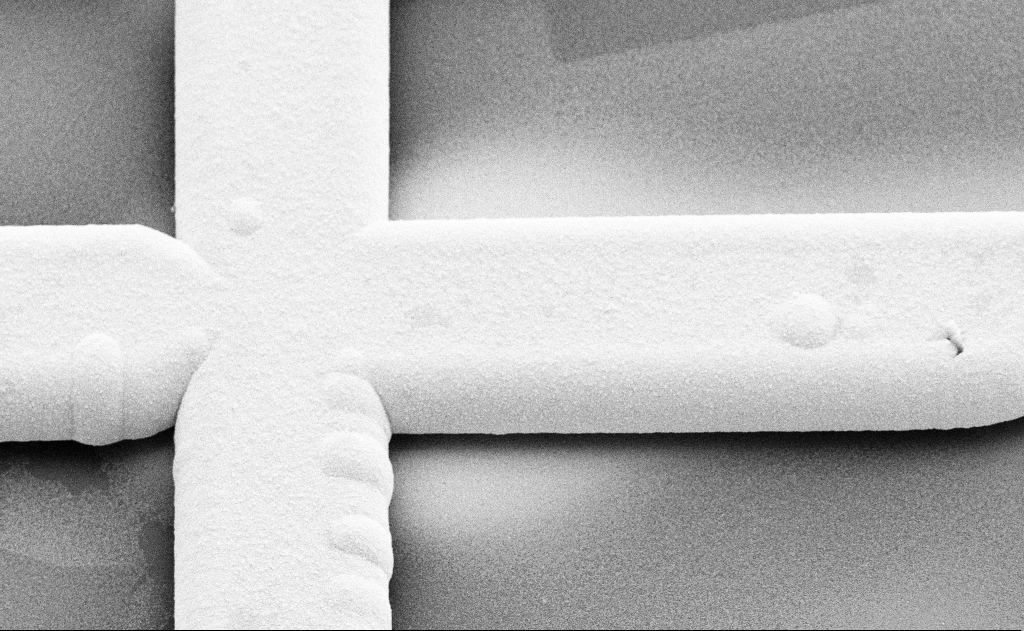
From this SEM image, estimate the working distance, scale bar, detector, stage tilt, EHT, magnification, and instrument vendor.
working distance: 8 mm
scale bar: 10000 nm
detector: SE2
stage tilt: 35°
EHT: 10 kV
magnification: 4.15 K X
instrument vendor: Zeiss